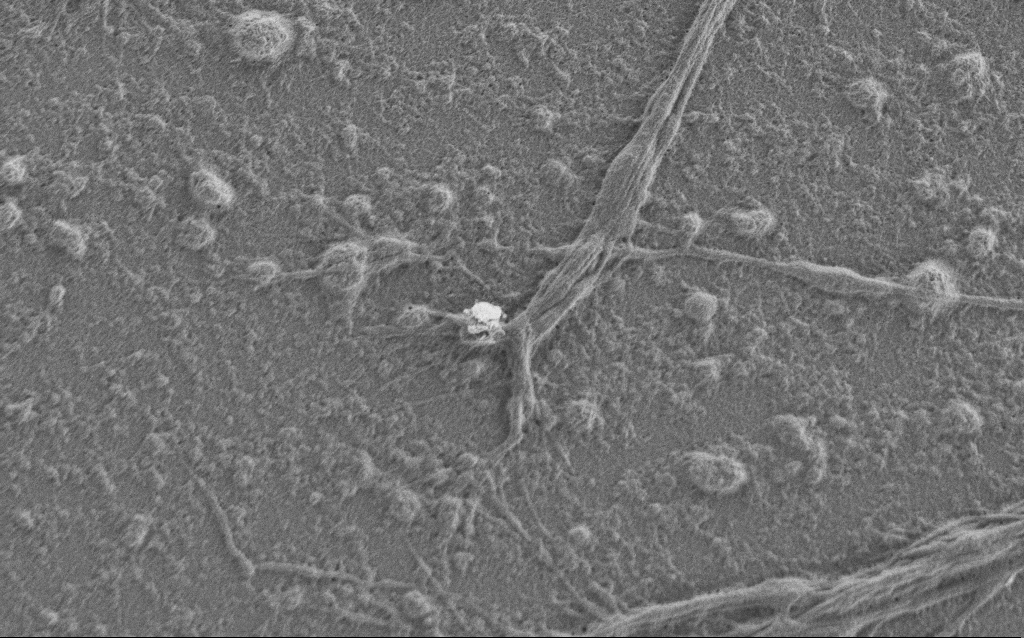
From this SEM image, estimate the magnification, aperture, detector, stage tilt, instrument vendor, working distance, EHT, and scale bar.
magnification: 7.5 K X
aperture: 30 µm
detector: SE2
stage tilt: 0°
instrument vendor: Zeiss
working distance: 6 mm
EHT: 1 kV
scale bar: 2000 nm